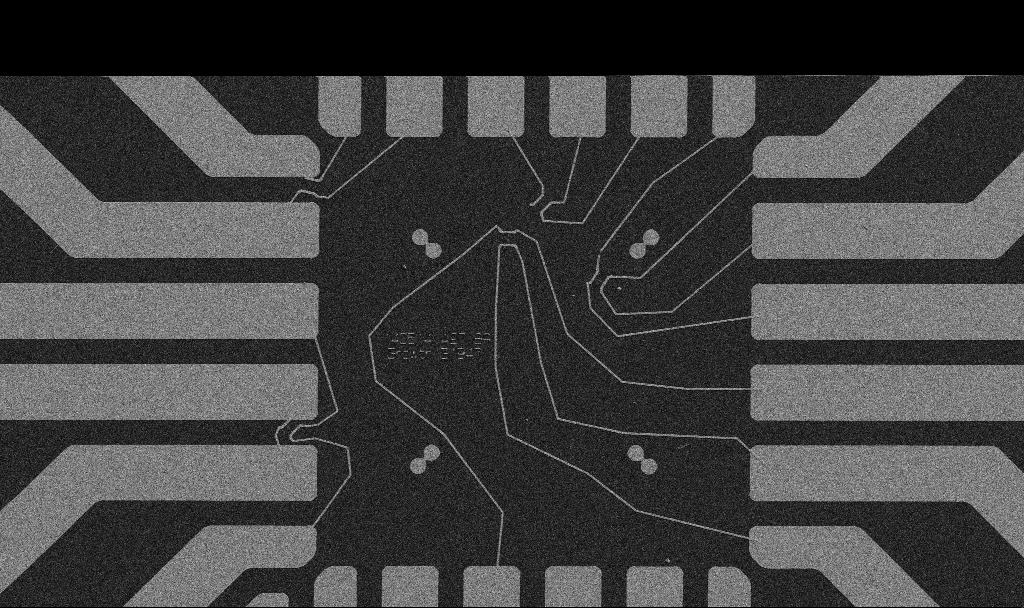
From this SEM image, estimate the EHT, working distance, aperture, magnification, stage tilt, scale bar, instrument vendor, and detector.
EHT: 5 kV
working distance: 10.7 mm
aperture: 30 µm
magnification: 1 K X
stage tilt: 0°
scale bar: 20000 nm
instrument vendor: Zeiss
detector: SE2